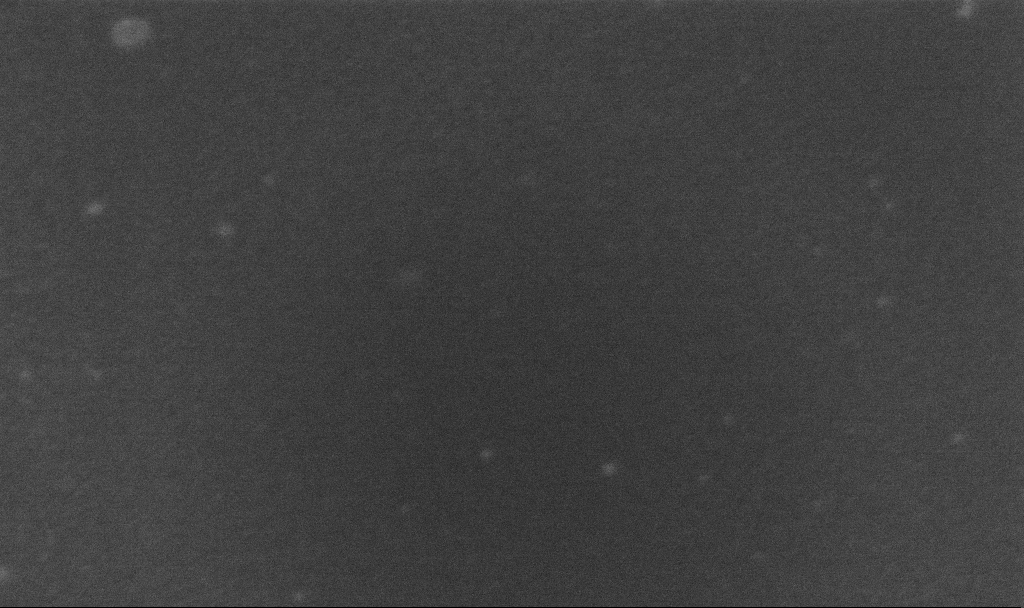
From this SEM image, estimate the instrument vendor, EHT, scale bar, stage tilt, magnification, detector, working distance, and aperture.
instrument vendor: Zeiss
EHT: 5 kV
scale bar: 100 nm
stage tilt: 0°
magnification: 500 K X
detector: InLens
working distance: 3.2 mm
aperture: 30 µm